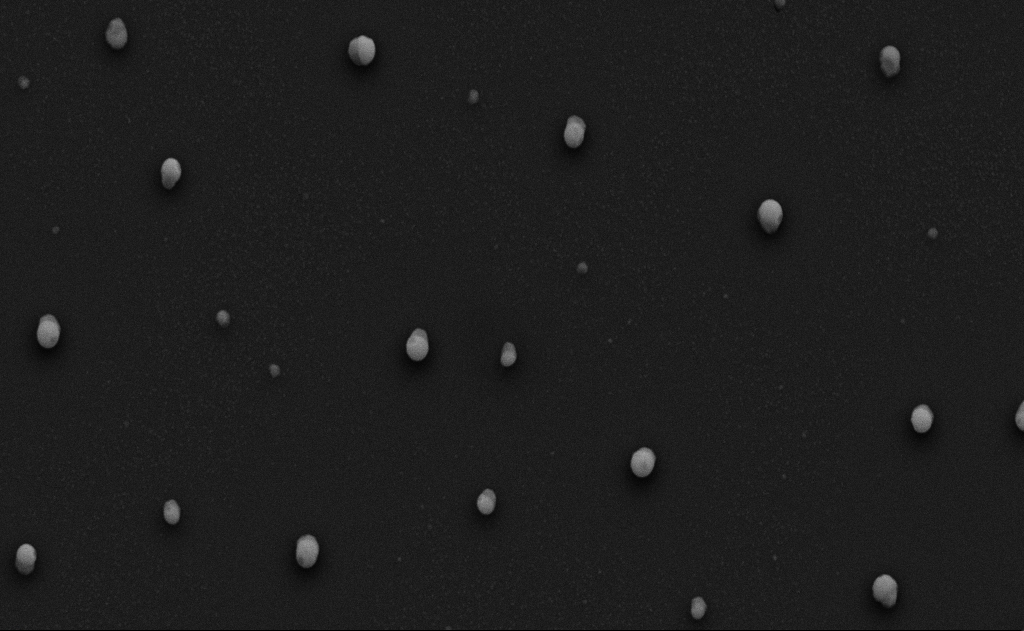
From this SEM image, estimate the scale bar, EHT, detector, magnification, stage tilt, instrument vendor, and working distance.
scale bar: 1000 nm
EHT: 10 kV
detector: SE2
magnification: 20 K X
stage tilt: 0°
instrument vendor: Zeiss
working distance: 9 mm